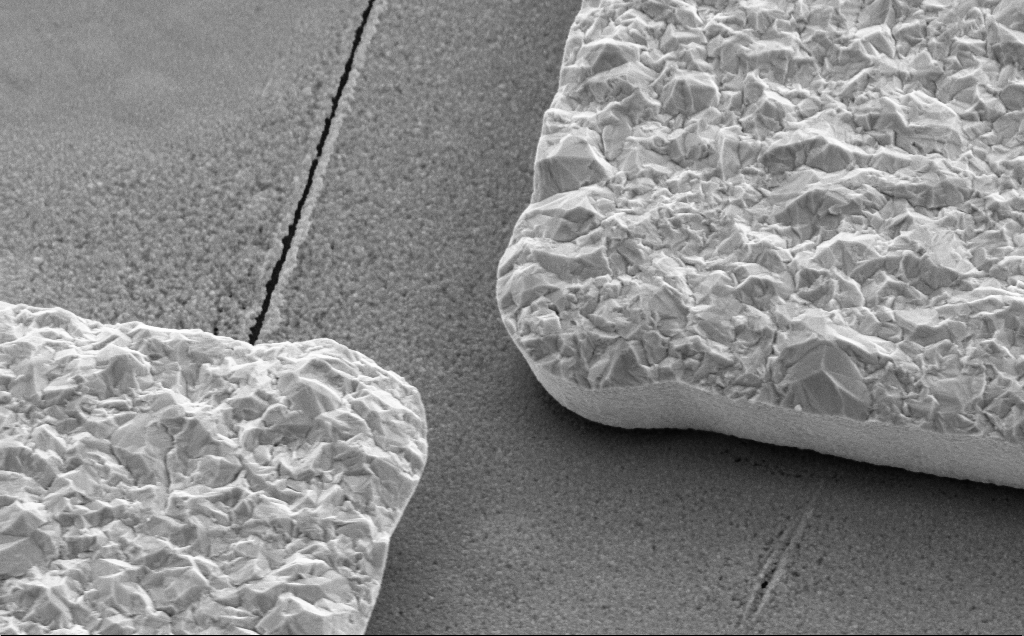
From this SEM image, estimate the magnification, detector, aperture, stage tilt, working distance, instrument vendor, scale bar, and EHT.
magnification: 33.34 K X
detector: SE2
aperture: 30 µm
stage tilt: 35°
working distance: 13 mm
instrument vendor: Zeiss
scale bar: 1000 nm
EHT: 5 kV